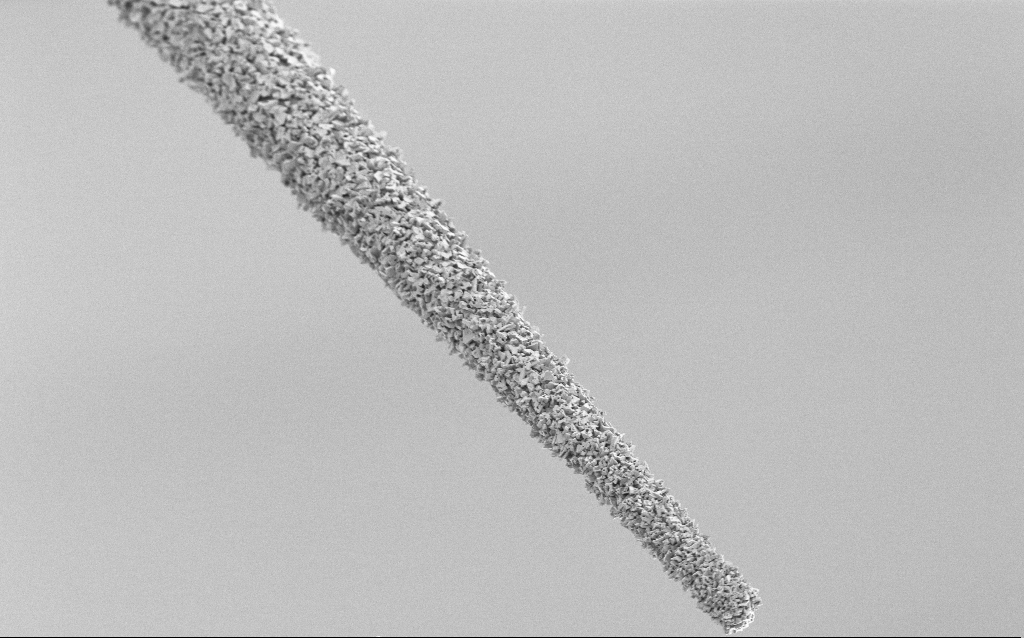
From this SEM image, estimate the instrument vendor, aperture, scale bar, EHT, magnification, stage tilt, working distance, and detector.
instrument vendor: Zeiss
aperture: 30 µm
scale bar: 10000 nm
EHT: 1.5 kV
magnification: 2.5 K X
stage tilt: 45°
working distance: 7.8 mm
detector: SE2